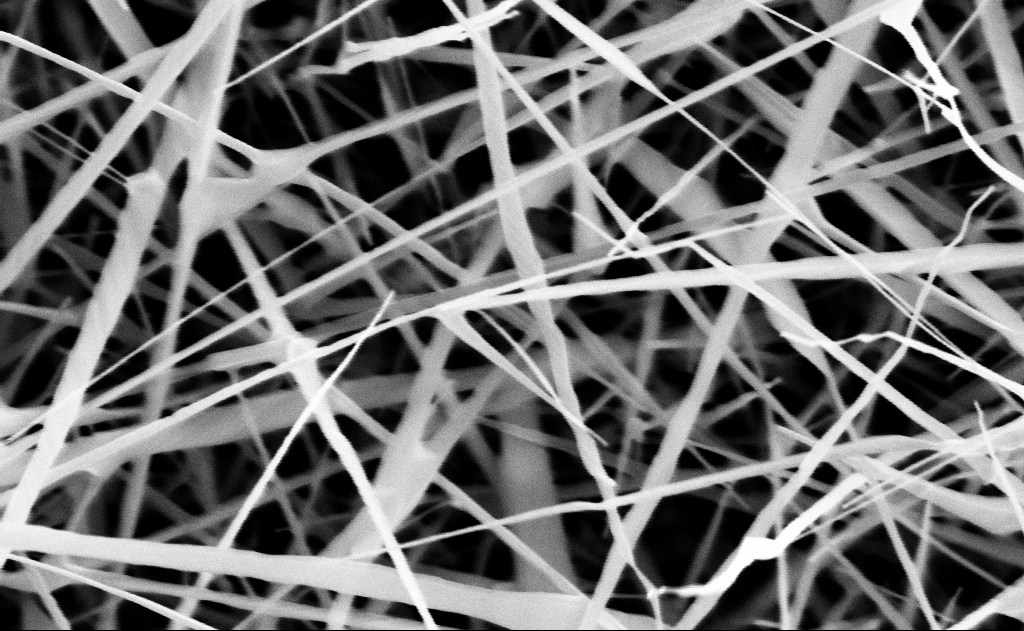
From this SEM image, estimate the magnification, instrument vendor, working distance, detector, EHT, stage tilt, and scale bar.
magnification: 80 K X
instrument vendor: Zeiss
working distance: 15 mm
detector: InLens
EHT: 10 kV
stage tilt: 0°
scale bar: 200 nm